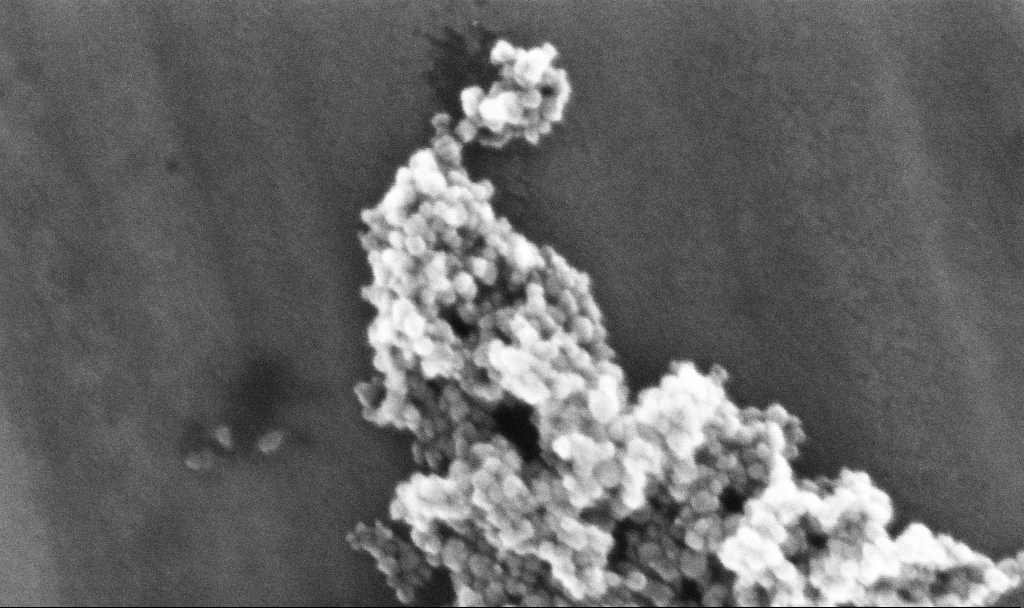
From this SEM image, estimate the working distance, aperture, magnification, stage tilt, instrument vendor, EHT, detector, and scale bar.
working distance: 5.3 mm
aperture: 30 µm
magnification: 370.56 K X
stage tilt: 0°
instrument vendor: Zeiss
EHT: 10 kV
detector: InLens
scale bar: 100 nm